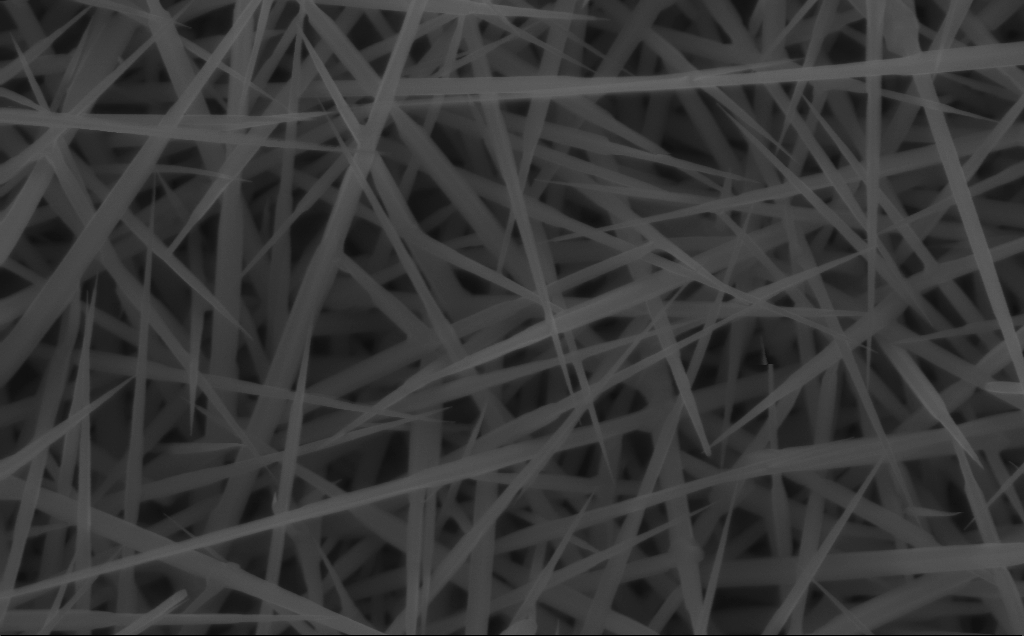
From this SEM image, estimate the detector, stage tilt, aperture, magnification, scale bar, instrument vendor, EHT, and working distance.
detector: InLens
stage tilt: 0°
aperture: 30 µm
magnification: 80 K X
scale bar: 200 nm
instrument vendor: Zeiss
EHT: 10 kV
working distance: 4 mm